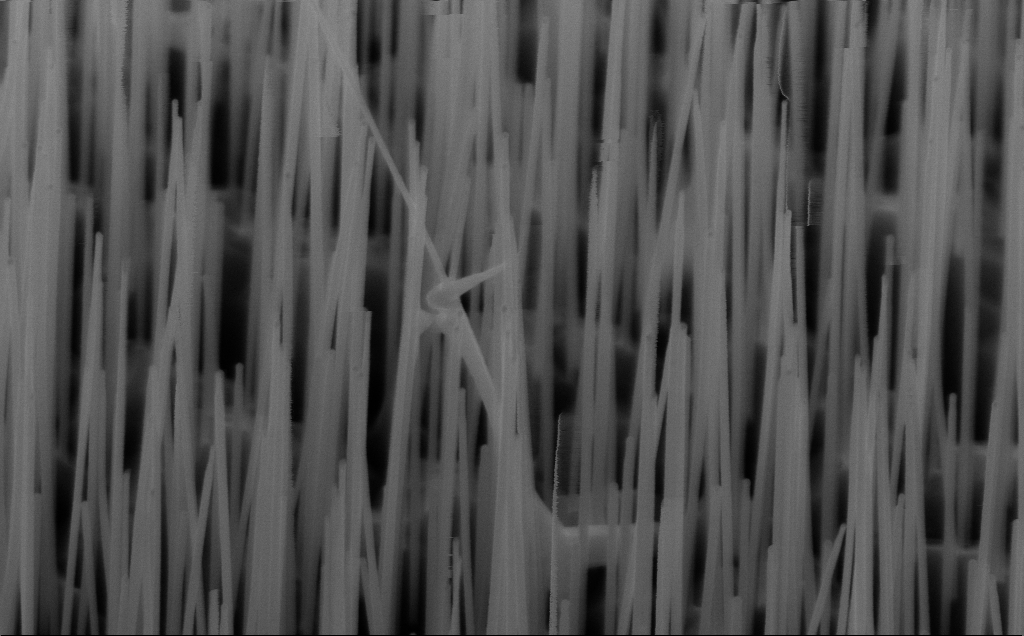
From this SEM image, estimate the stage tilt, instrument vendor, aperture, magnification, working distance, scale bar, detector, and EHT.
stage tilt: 45°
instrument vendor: Zeiss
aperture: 30 µm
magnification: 80 K X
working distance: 5 mm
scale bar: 200 nm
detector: InLens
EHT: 10 kV